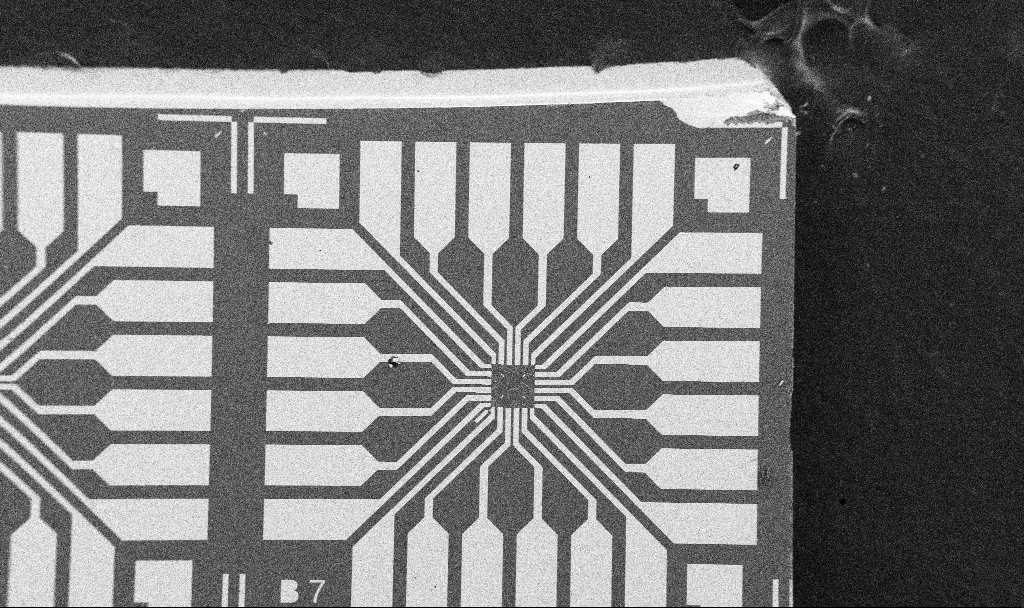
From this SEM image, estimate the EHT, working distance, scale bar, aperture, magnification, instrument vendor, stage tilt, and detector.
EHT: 5 kV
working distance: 10.7 mm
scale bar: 200000 nm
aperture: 30 µm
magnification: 0.1 K X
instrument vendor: Zeiss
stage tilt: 0°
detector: SE2